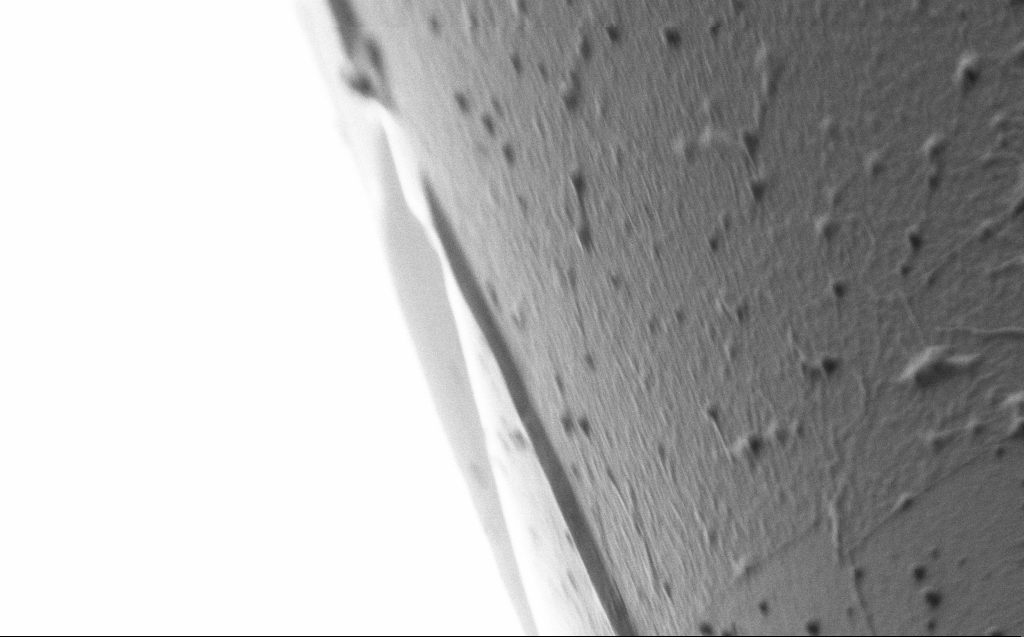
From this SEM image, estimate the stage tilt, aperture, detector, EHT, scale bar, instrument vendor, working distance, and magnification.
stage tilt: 45°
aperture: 30 µm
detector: SE2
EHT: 1 kV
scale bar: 2000 nm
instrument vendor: Zeiss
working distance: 4 mm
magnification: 27.61 K X